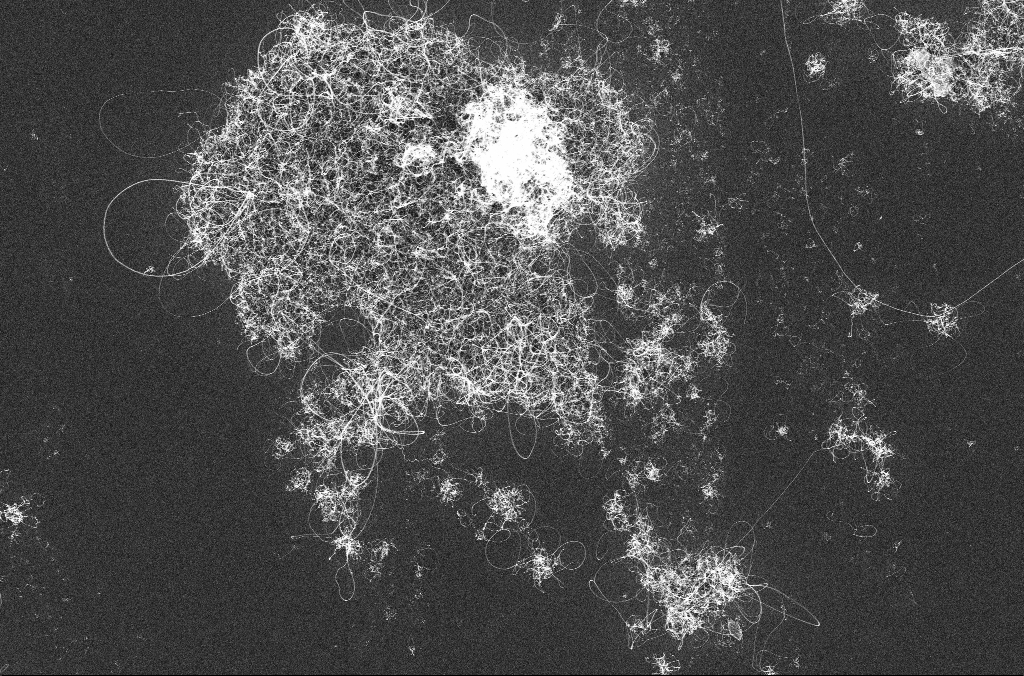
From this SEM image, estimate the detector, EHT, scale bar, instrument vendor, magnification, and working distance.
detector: InLens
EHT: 10 kV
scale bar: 2000 nm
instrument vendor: Zeiss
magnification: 10 K X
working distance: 3.3 mm